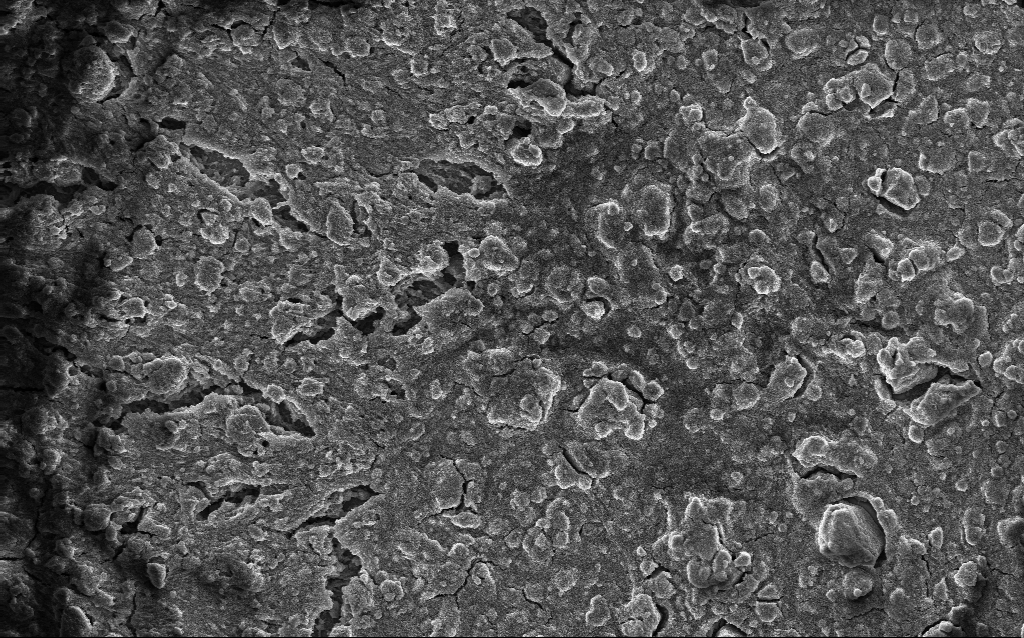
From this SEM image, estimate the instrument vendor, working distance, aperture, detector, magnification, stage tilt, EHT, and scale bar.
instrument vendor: Zeiss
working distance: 2.6 mm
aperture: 30 µm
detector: InLens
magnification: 1.69 K X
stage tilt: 0°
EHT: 10 kV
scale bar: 10000 nm